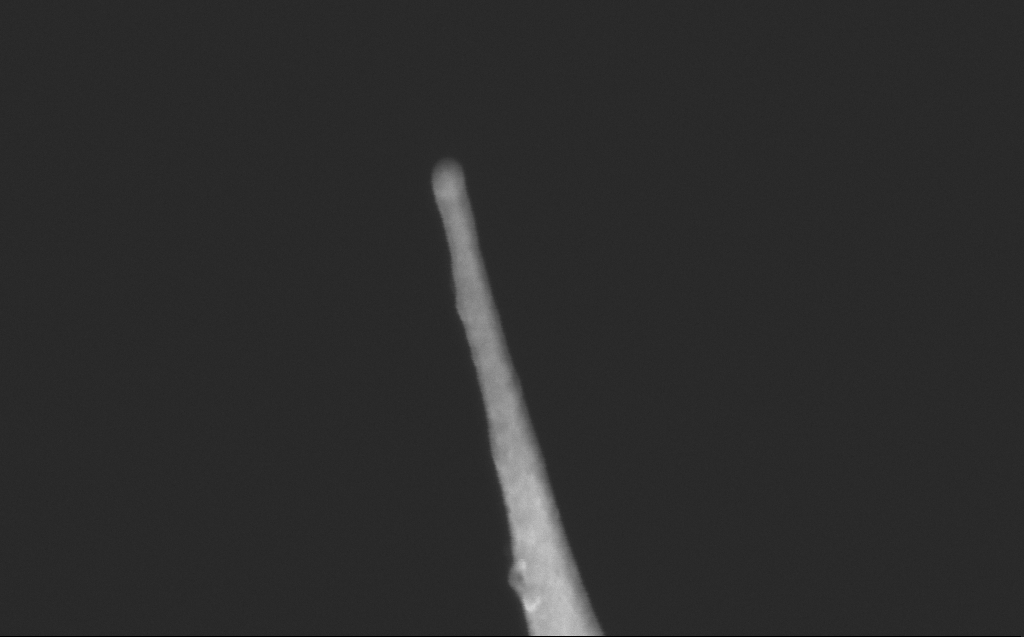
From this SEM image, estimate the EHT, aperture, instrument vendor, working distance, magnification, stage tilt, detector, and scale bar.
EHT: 10 kV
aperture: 30 µm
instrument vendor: Zeiss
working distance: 6 mm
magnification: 220.66 K X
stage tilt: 40°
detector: InLens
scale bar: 100 nm